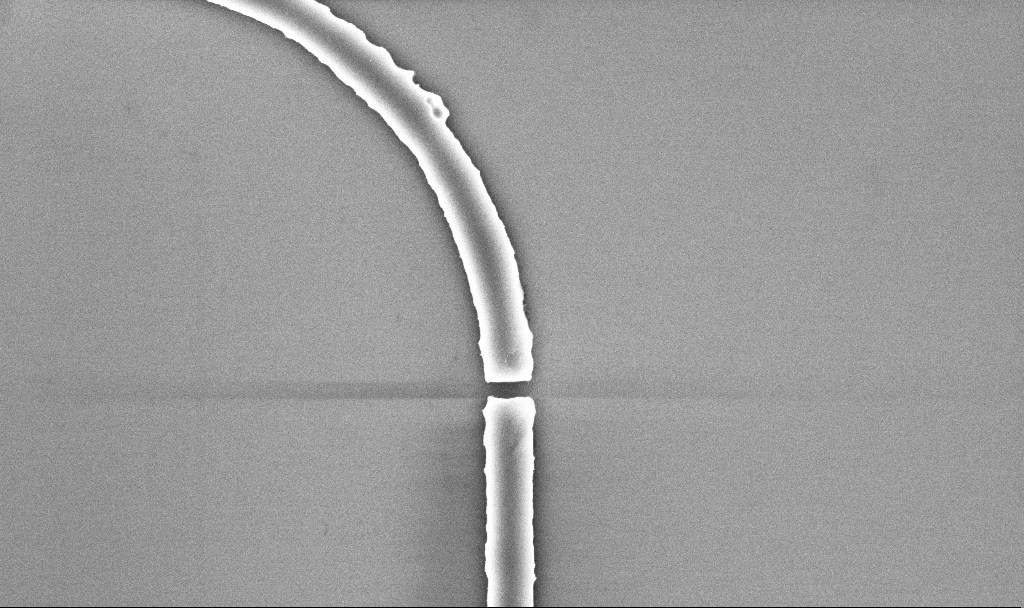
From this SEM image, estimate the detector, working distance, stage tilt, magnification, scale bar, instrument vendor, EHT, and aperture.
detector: InLens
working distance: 5.2 mm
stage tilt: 0°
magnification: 34.88 K X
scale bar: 1000 nm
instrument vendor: Zeiss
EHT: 5 kV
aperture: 30 µm